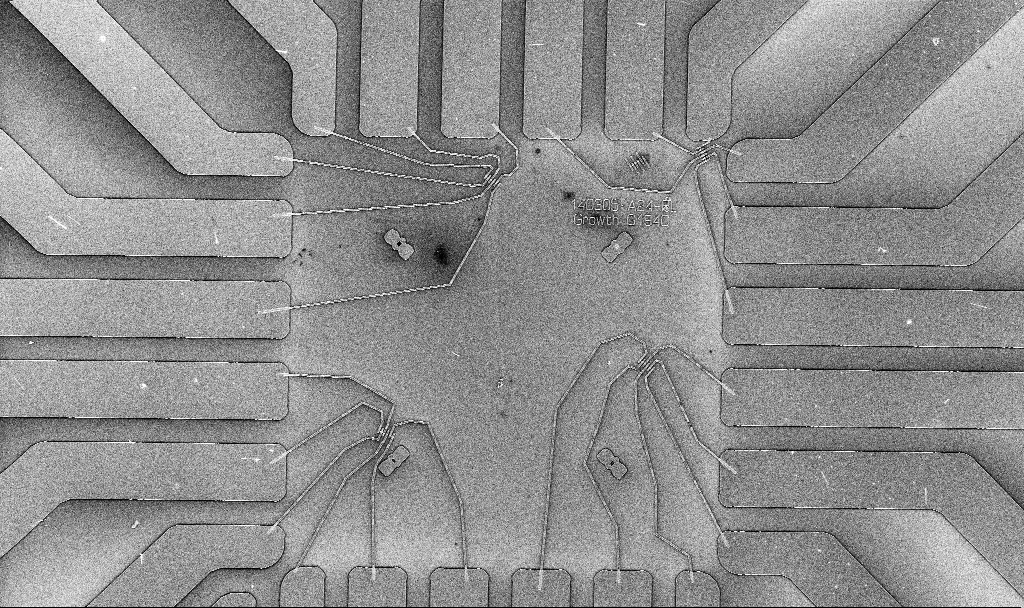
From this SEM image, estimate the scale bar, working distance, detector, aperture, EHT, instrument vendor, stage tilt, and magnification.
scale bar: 20000 nm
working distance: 6.8 mm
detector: InLens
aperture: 30 µm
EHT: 10 kV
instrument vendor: Zeiss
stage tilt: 0°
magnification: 1 K X